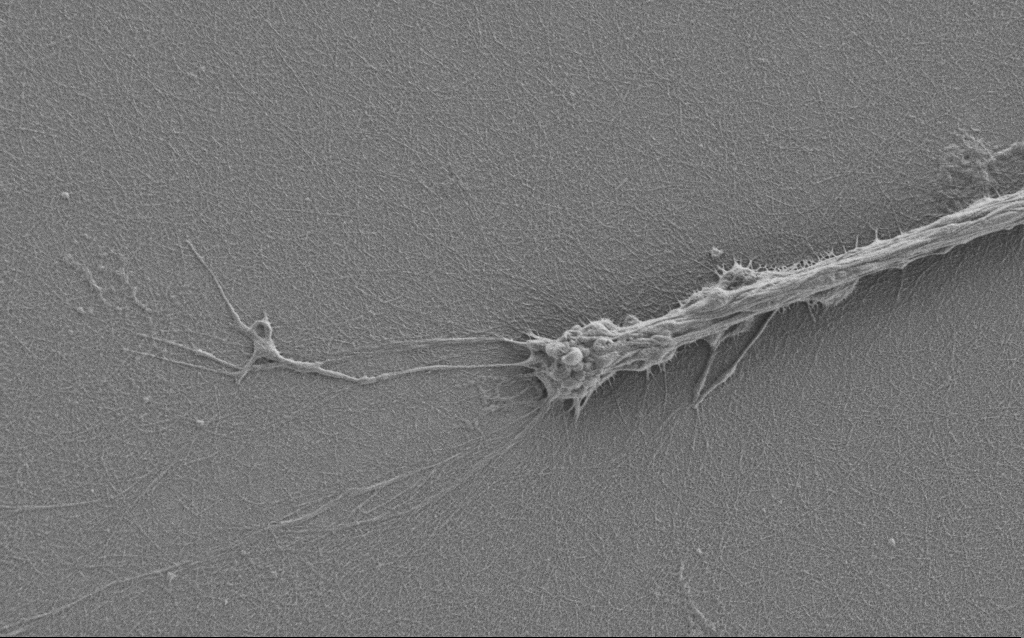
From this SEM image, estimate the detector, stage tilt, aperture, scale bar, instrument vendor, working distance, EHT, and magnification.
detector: SE2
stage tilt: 0°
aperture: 30 µm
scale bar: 2000 nm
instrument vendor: Zeiss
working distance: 6 mm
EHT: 1 kV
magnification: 7.5 K X